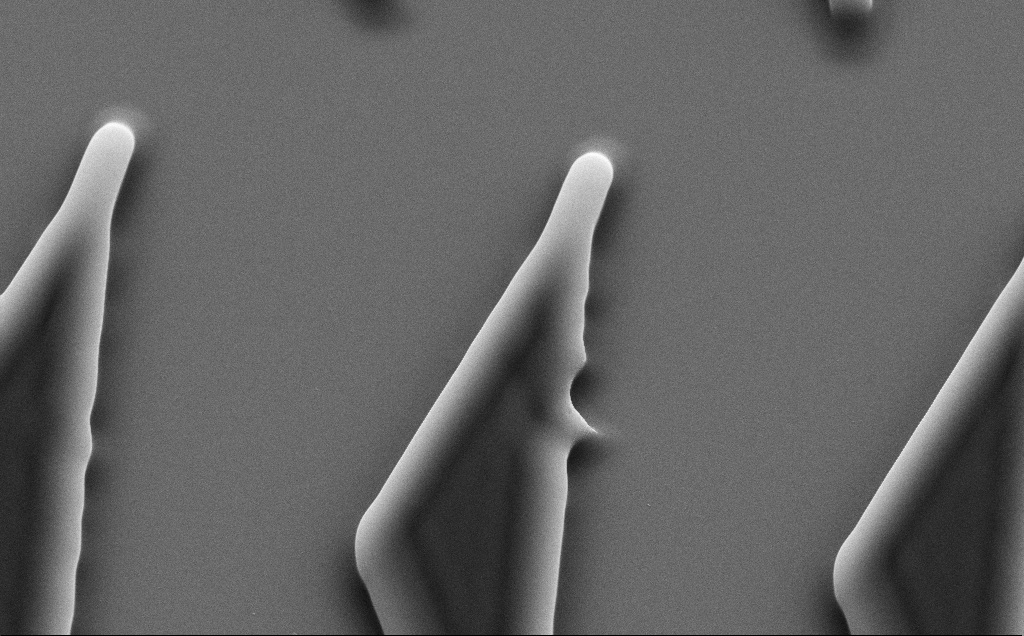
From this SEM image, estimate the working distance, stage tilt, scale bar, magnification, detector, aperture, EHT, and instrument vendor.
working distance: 8 mm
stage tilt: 35°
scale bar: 2000 nm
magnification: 10.39 K X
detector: SE2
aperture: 30 µm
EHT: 10 kV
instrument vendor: Zeiss